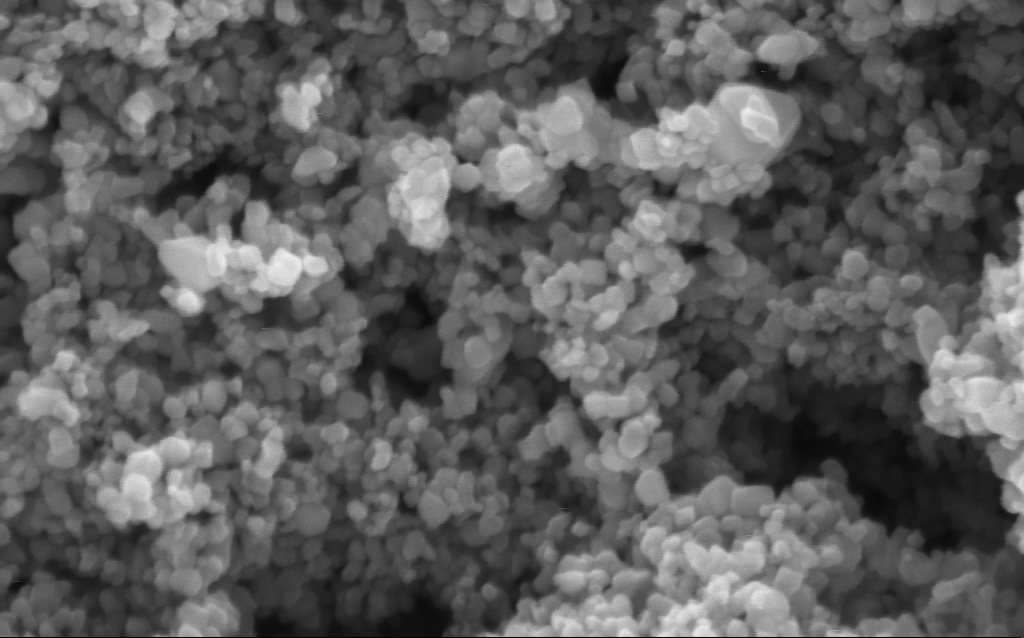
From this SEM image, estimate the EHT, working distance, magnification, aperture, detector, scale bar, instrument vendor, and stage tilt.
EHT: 5 kV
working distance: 4.7 mm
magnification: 290.6 K X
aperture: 30 µm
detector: InLens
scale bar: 200 nm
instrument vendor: Zeiss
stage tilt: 0°